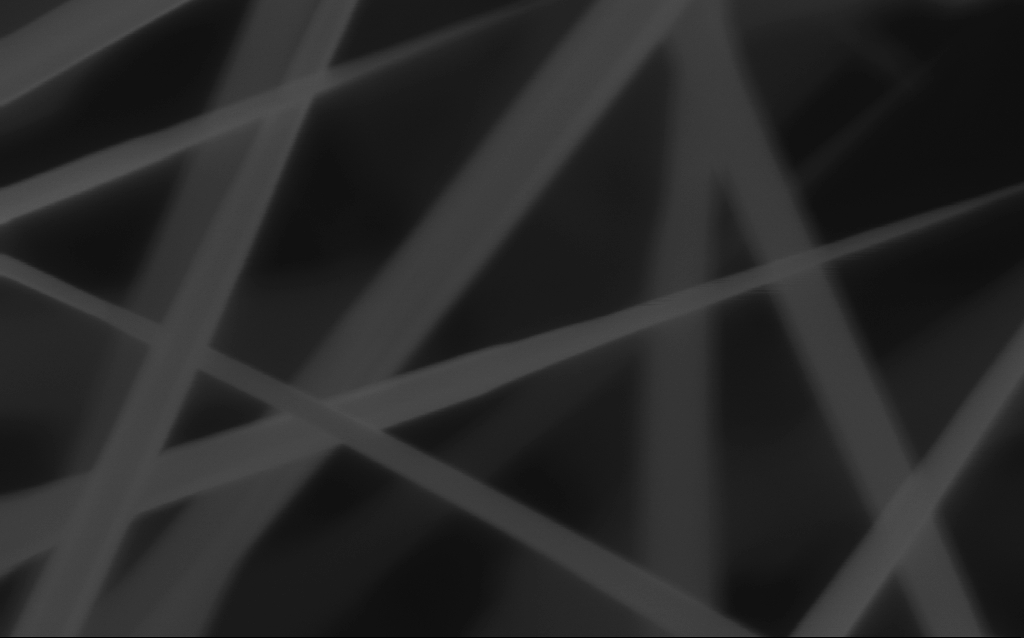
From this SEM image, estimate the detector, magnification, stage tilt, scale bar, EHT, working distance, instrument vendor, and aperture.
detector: InLens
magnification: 200 K X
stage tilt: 0°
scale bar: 100 nm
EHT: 10 kV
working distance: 6 mm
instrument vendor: Zeiss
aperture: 30 µm